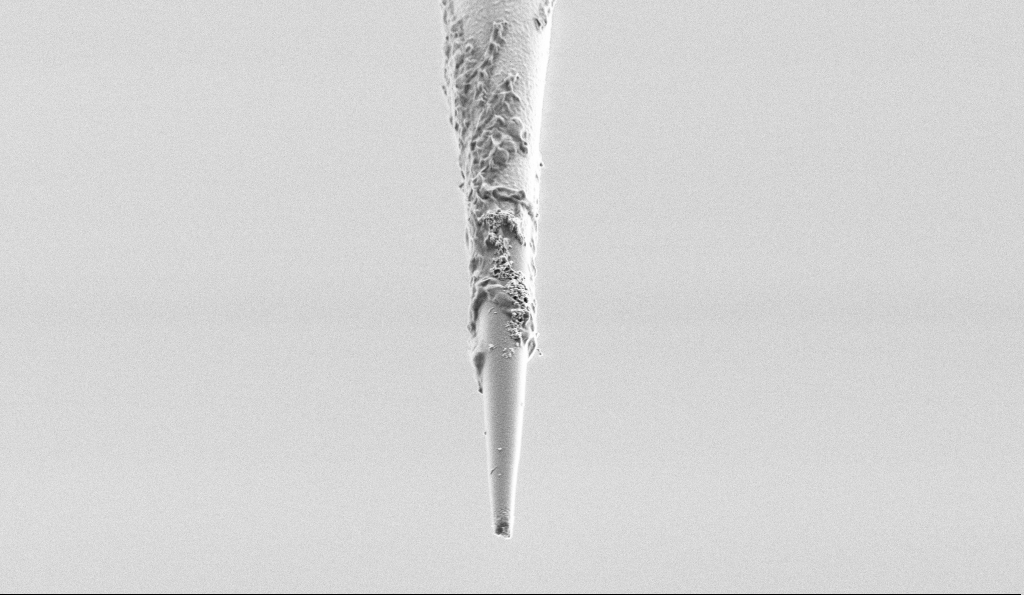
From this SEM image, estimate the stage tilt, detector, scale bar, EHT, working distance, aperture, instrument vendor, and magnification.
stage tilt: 45°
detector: SE2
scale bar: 2000 nm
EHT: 1 kV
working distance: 7.4 mm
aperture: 30 µm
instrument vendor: Zeiss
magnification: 15 K X